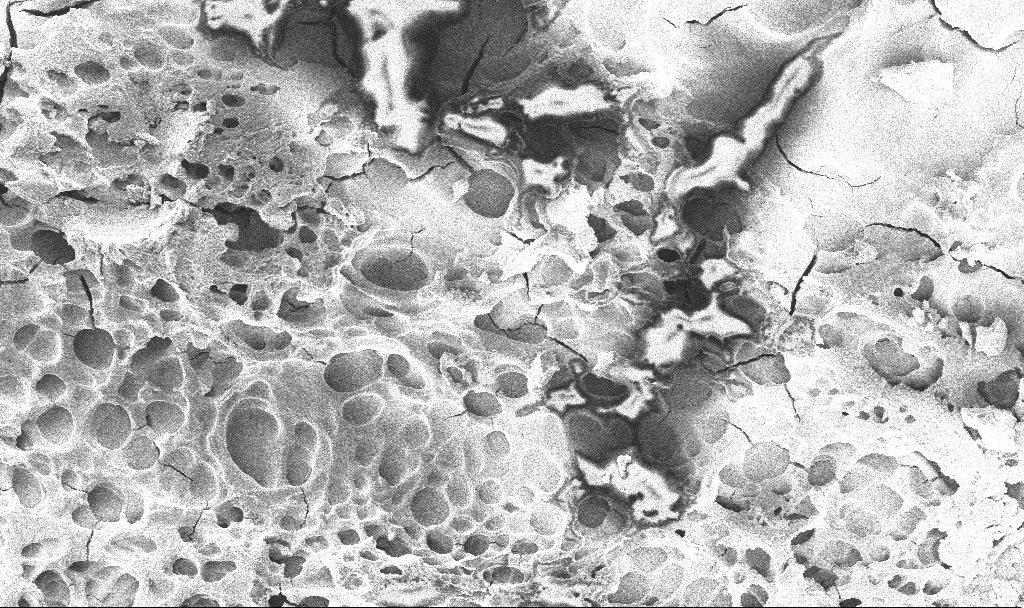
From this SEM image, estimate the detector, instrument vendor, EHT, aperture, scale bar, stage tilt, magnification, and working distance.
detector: InLens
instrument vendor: Zeiss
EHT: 3 kV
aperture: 30 µm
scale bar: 100000 nm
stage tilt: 0°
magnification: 0.672 K X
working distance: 2.5 mm